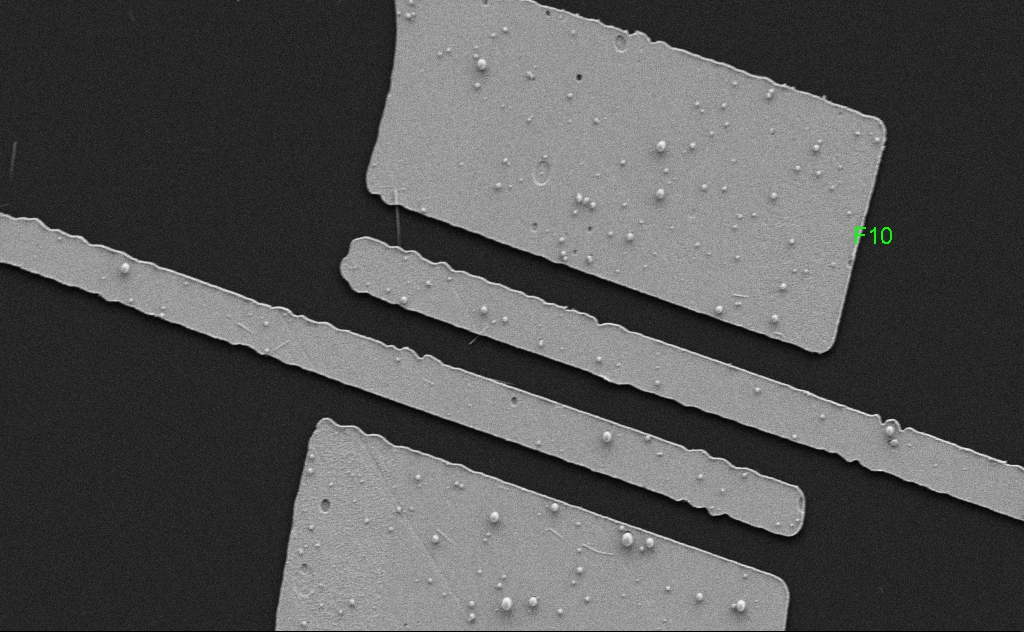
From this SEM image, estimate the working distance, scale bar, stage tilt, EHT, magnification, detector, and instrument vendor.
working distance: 5 mm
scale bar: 10000 nm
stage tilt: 0°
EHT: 5 kV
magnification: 6.43 K X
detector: SE2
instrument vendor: Zeiss